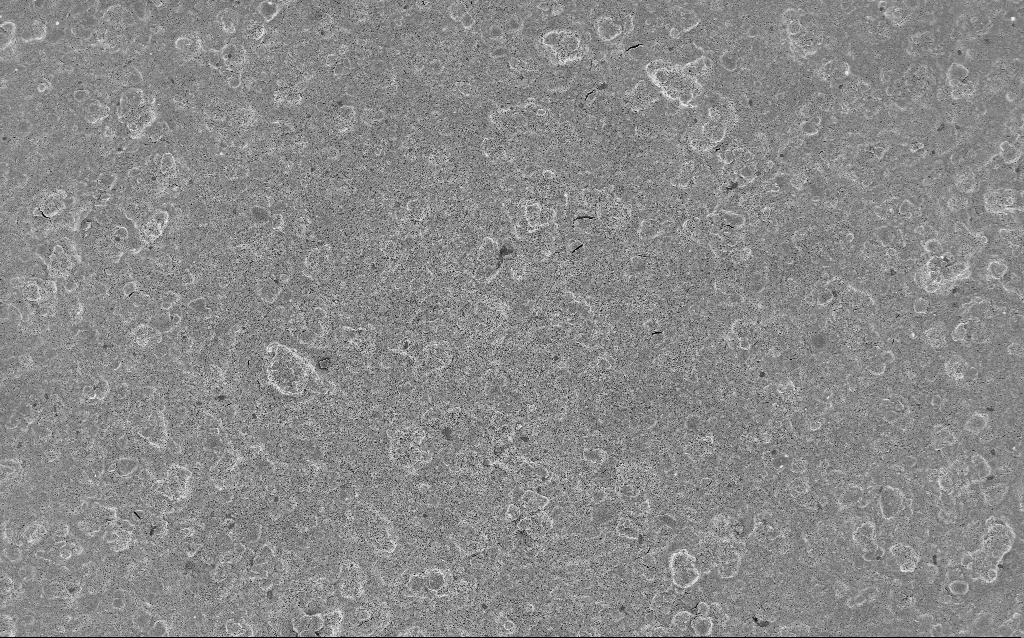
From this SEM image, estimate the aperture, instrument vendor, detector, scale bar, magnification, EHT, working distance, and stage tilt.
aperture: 30 µm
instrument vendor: Zeiss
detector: InLens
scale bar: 20000 nm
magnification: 0.77 K X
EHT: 3 kV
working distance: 7.5 mm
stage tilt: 0°